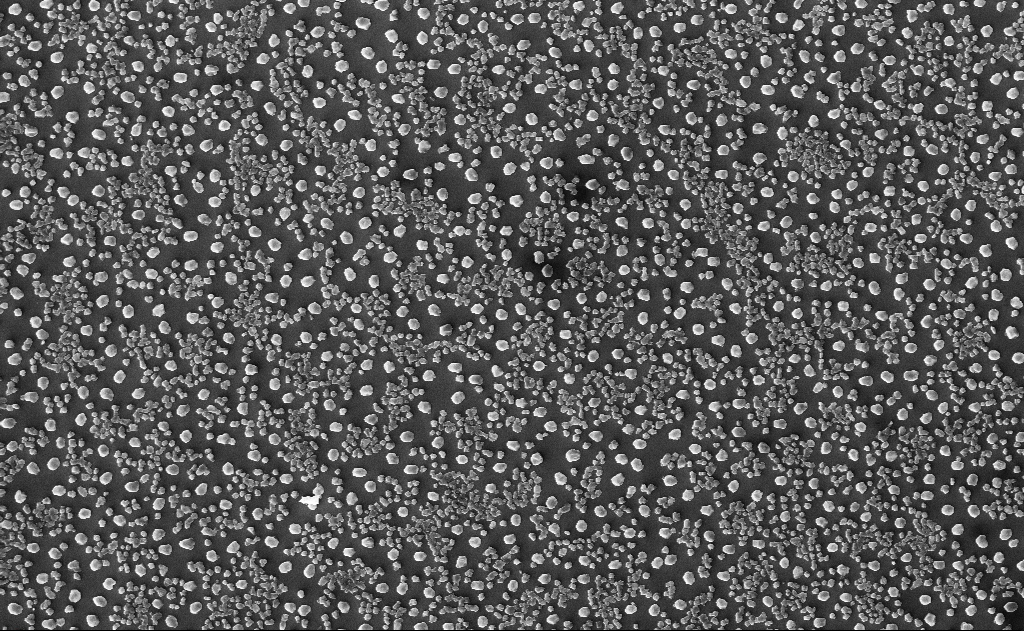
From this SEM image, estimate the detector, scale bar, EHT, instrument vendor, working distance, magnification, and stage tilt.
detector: InLens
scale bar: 2000 nm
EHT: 10 kV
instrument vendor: Zeiss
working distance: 16 mm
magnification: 10 K X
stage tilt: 0°